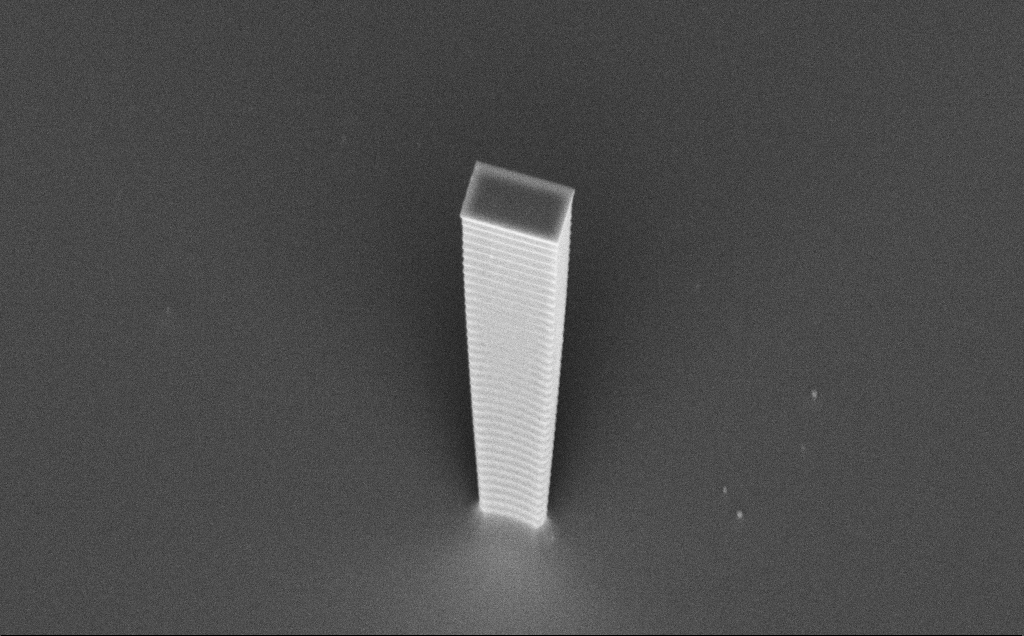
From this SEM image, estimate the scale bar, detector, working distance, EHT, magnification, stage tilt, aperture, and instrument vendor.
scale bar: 2000 nm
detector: InLens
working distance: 8 mm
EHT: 7.5 kV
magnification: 7.8 K X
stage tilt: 45°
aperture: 30 µm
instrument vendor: Zeiss